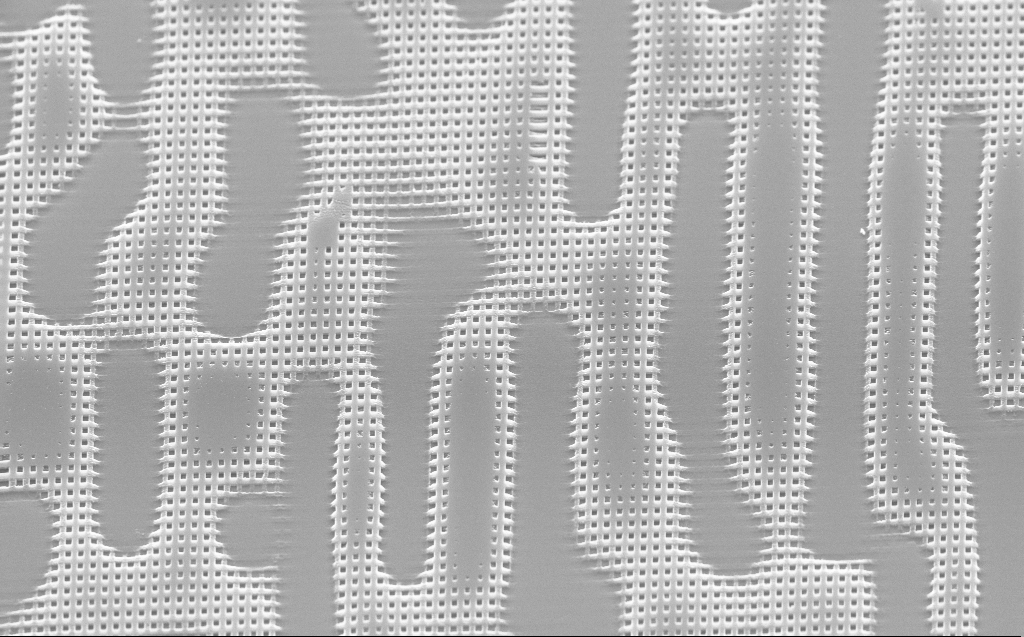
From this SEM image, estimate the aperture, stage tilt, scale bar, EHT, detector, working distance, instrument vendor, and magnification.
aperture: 30 µm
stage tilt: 45°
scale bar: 2000 nm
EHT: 10 kV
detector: InLens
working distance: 3 mm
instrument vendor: Zeiss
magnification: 9.31 K X